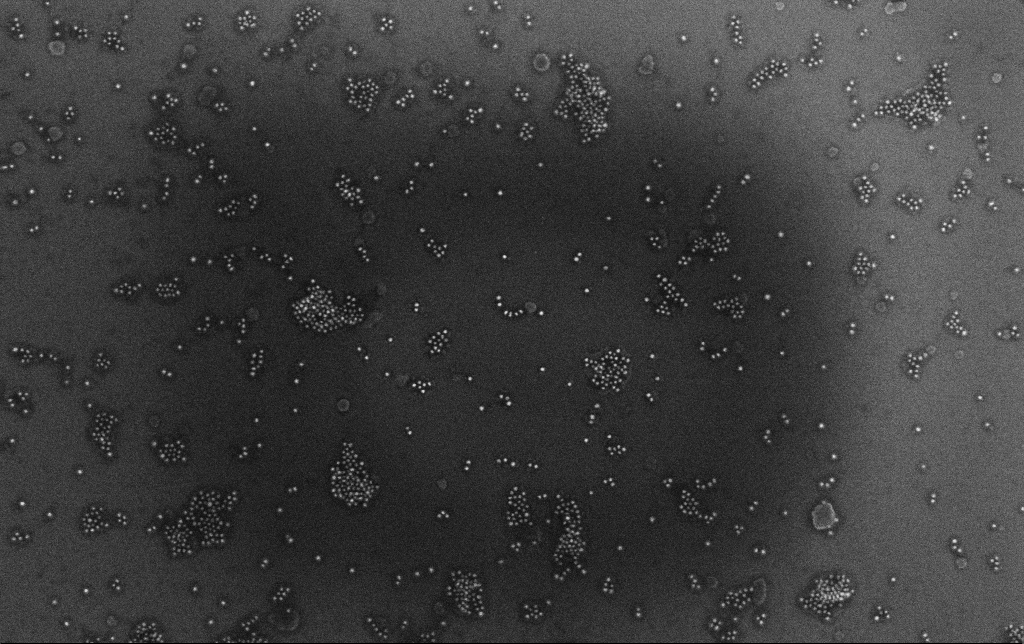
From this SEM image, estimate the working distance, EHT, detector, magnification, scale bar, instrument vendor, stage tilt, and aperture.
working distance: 3.4 mm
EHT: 10 kV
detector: InLens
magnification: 100 K X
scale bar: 200 nm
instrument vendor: Zeiss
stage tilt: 0°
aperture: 30 µm